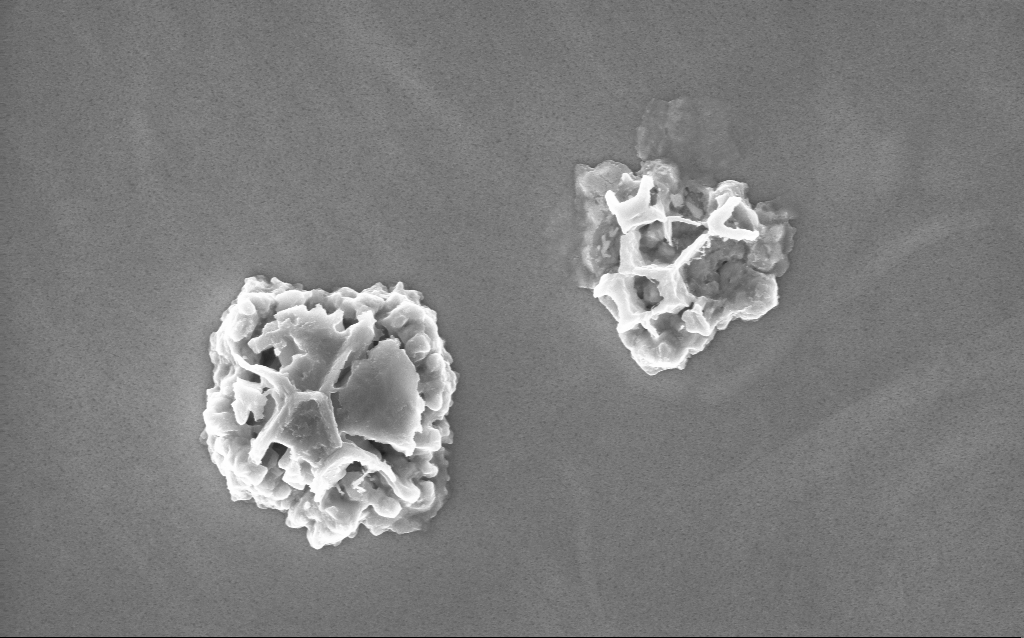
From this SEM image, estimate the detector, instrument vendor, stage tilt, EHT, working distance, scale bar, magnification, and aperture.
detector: InLens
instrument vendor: Zeiss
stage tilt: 0°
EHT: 10 kV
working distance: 2 mm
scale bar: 1000 nm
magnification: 46.82 K X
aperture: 30 µm